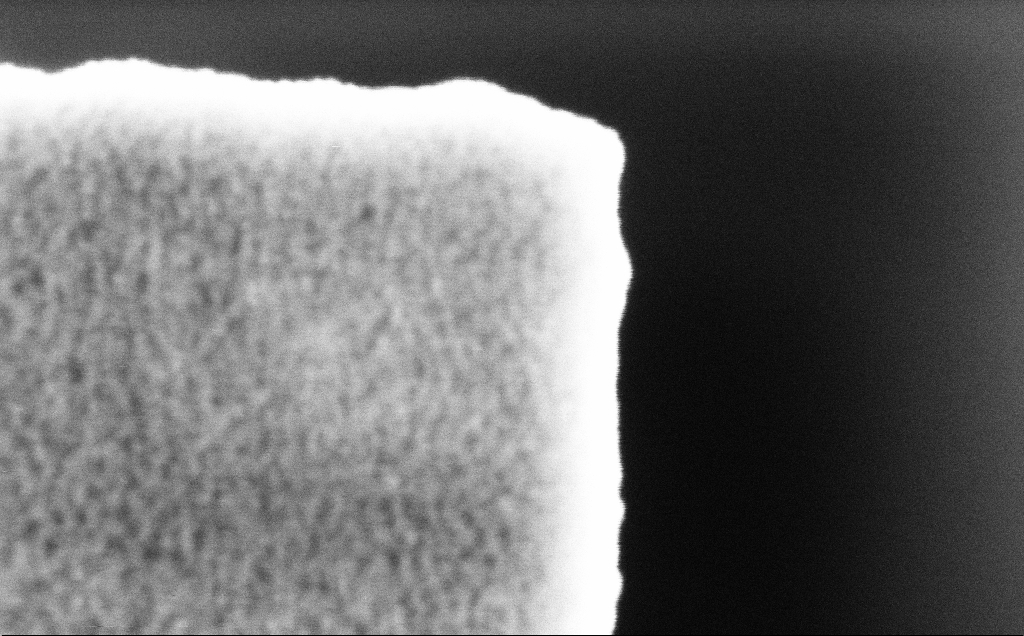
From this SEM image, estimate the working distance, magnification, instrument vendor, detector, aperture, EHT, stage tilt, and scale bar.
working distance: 3.2 mm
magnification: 333.13 K X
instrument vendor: Zeiss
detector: InLens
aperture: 30 µm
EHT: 3 kV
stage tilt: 0°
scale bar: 100 nm